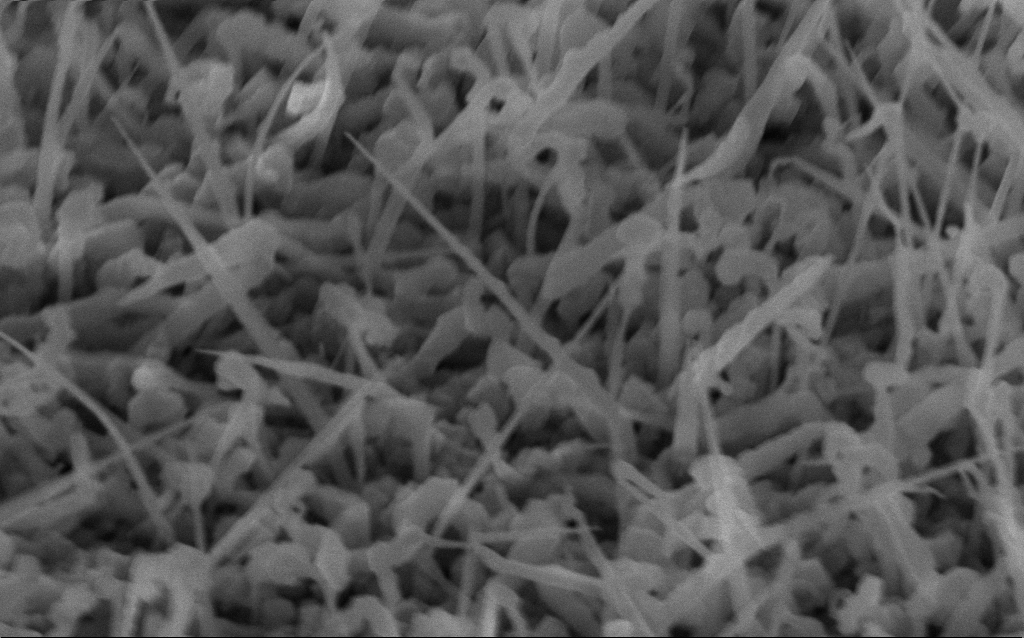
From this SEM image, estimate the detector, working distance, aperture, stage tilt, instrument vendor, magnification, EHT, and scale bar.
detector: InLens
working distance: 3 mm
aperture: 30 µm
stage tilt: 53°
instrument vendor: Zeiss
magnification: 105 K X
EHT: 10 kV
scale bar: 200 nm